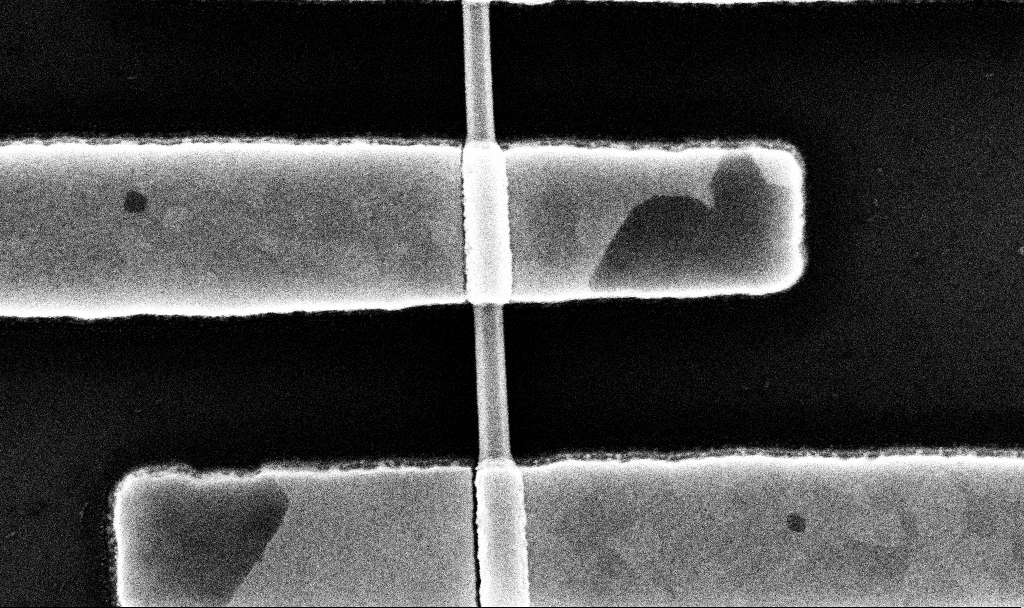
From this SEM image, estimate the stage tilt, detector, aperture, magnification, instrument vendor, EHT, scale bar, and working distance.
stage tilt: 0°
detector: InLens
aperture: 30 µm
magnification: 98.91 K X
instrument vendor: Zeiss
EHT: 10 kV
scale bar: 200 nm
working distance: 6.8 mm